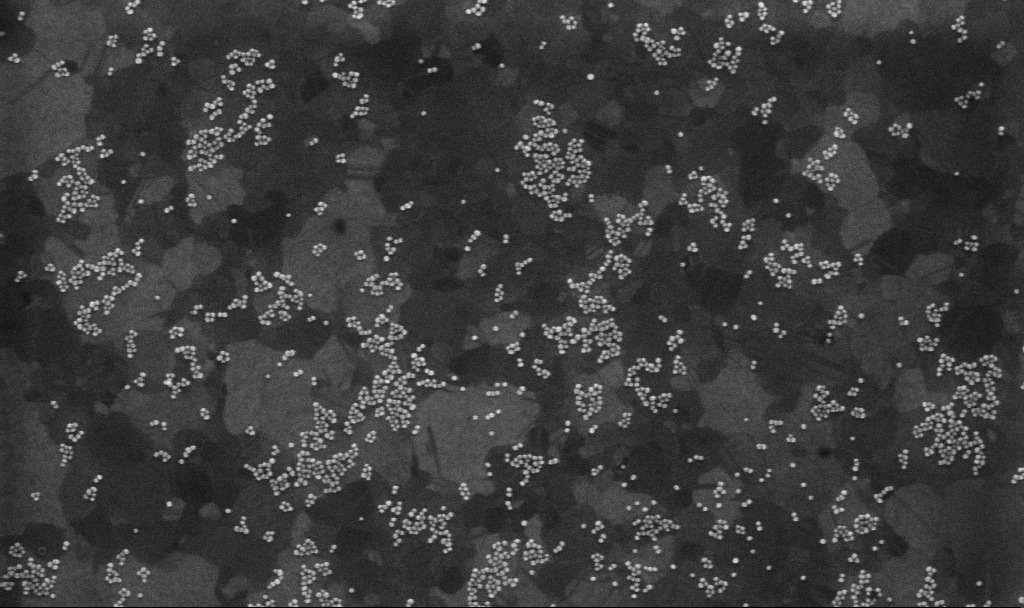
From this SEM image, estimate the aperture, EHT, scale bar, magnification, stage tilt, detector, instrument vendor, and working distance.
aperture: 30 µm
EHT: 10 kV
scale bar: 200 nm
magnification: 100 K X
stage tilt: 0°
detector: InLens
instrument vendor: Zeiss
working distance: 3.8 mm